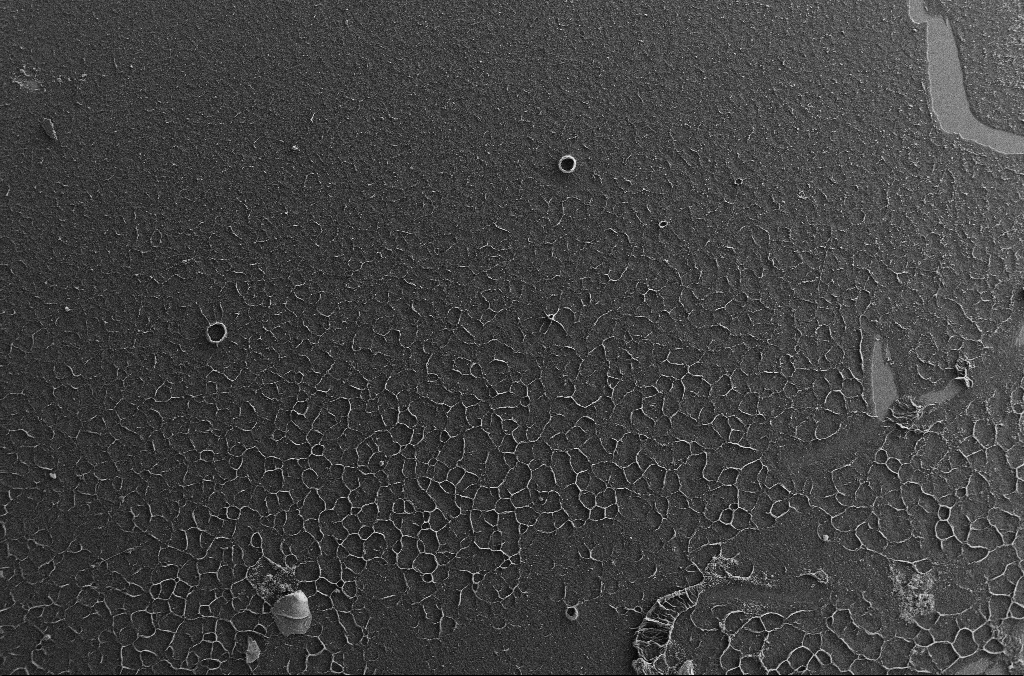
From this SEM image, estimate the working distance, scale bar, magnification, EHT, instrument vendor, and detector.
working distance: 2.9 mm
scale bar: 100000 nm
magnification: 0.15 K X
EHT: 7 kV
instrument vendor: Zeiss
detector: SE2